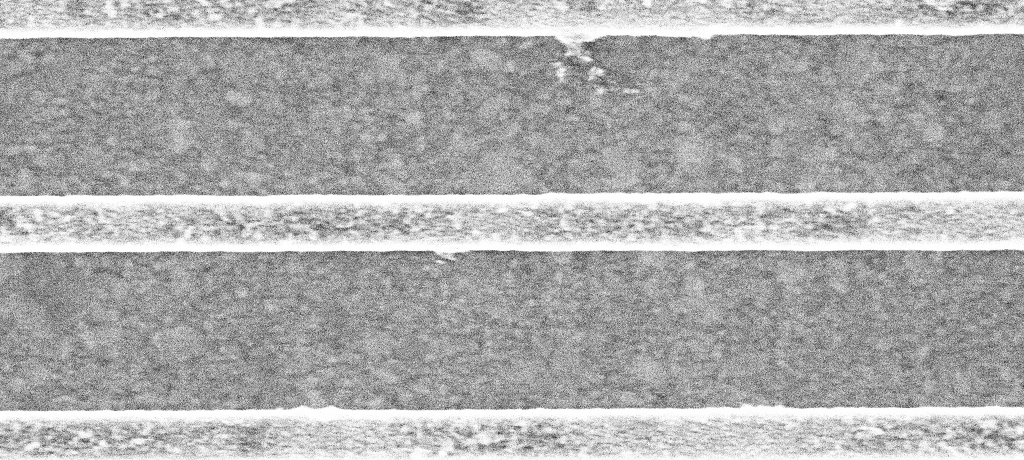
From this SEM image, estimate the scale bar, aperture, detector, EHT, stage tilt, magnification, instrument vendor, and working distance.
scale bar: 200 nm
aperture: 30 µm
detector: InLens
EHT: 5 kV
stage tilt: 0°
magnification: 99.68 K X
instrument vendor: Zeiss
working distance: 3.1 mm